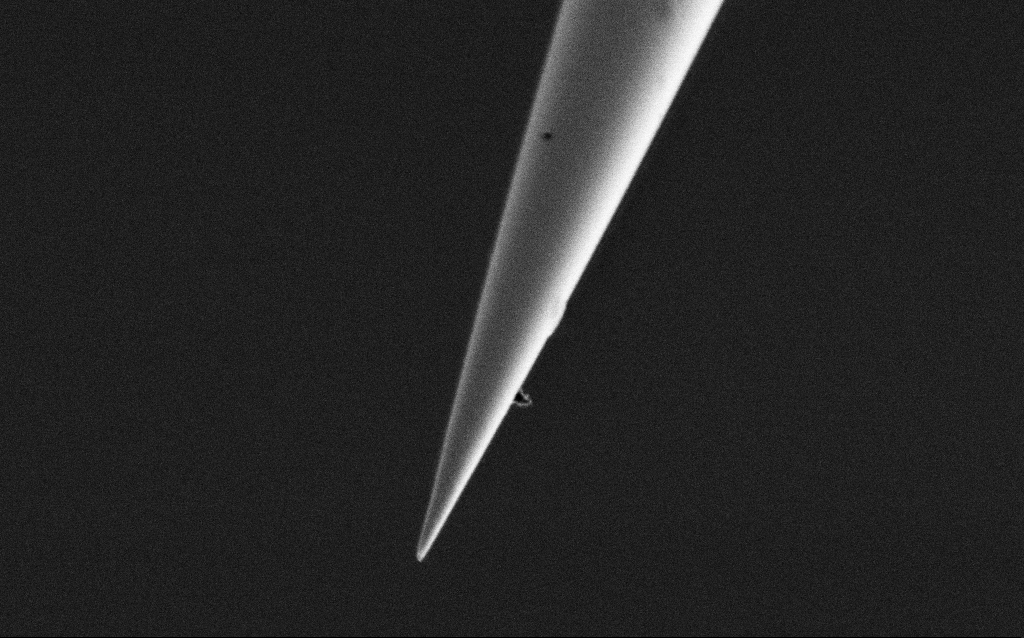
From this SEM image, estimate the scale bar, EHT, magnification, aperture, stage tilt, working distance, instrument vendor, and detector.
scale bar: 2000 nm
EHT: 1 kV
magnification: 25 K X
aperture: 30 µm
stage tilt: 45°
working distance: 7.4 mm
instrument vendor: Zeiss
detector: SE2